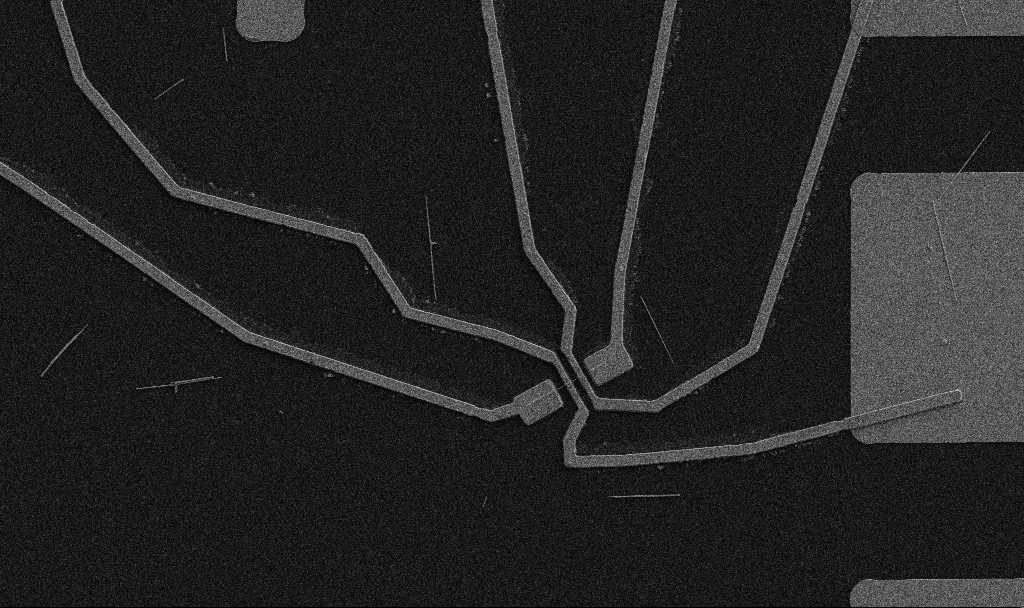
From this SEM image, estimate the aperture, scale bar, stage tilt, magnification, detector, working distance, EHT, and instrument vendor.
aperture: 30 µm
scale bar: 10000 nm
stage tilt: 0°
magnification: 5 K X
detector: SE2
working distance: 10.7 mm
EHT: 5 kV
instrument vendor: Zeiss